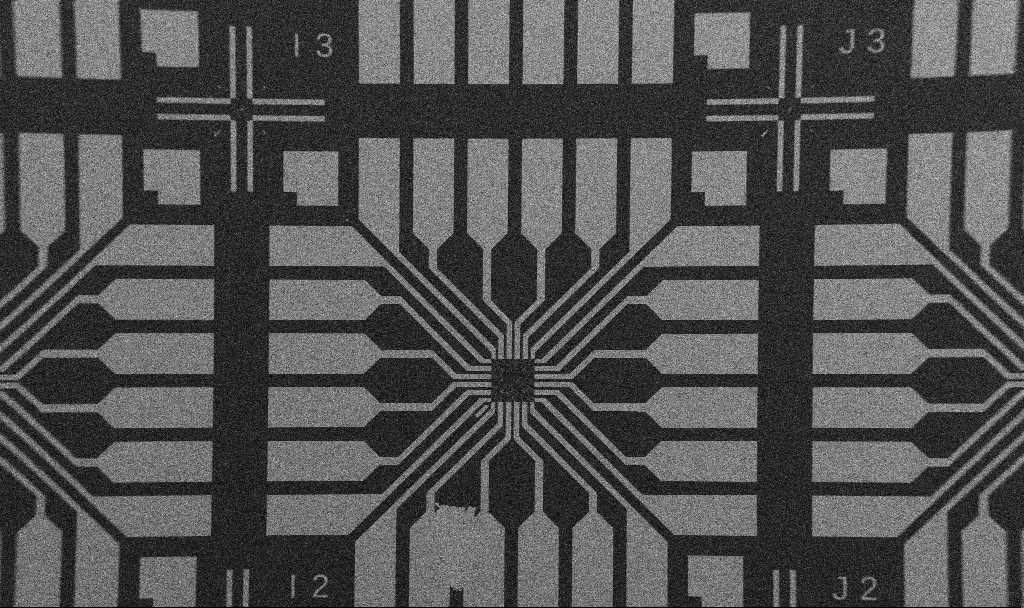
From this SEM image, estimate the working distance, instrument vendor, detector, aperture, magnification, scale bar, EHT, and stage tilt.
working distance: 10.7 mm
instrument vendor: Zeiss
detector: SE2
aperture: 30 µm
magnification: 0.1 K X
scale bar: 200000 nm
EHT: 5 kV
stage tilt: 0°